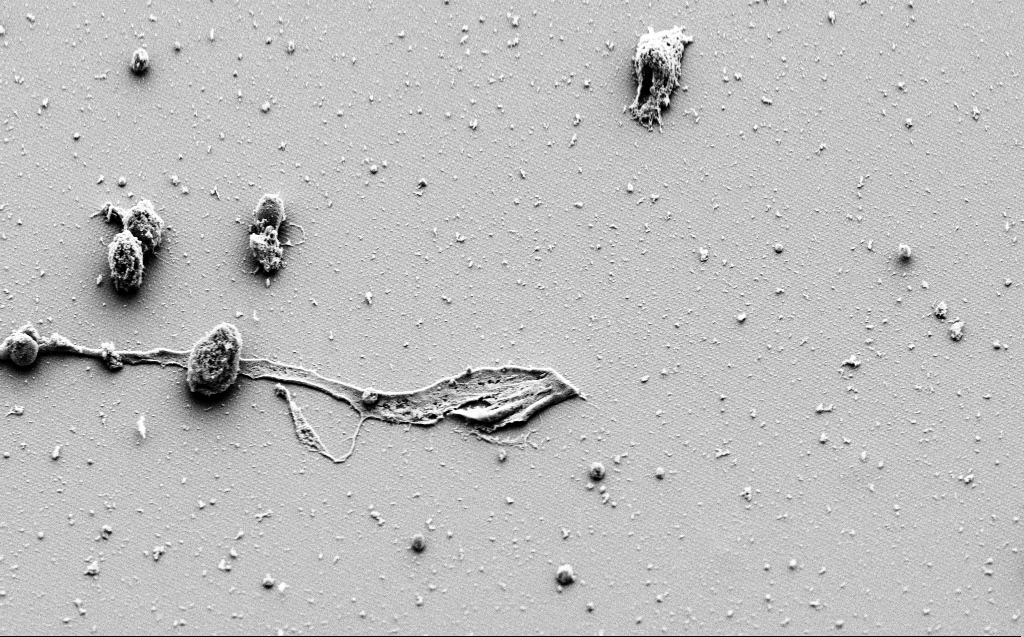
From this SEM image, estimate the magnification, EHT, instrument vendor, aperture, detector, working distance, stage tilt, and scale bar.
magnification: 2.93 K X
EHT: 3 kV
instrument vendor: Zeiss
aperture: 30 µm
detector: SE2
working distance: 9 mm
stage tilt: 45°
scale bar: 20000 nm